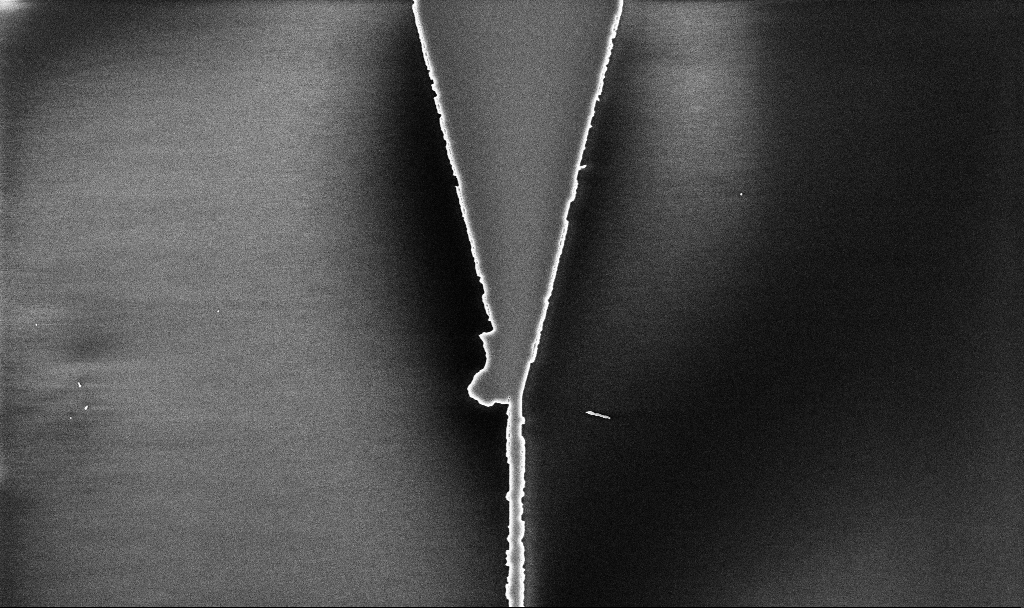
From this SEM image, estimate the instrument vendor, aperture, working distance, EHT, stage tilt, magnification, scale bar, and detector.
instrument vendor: Zeiss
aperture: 30 µm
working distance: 10.1 mm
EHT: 5 kV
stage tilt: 0°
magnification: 8.51 K X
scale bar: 2000 nm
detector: InLens